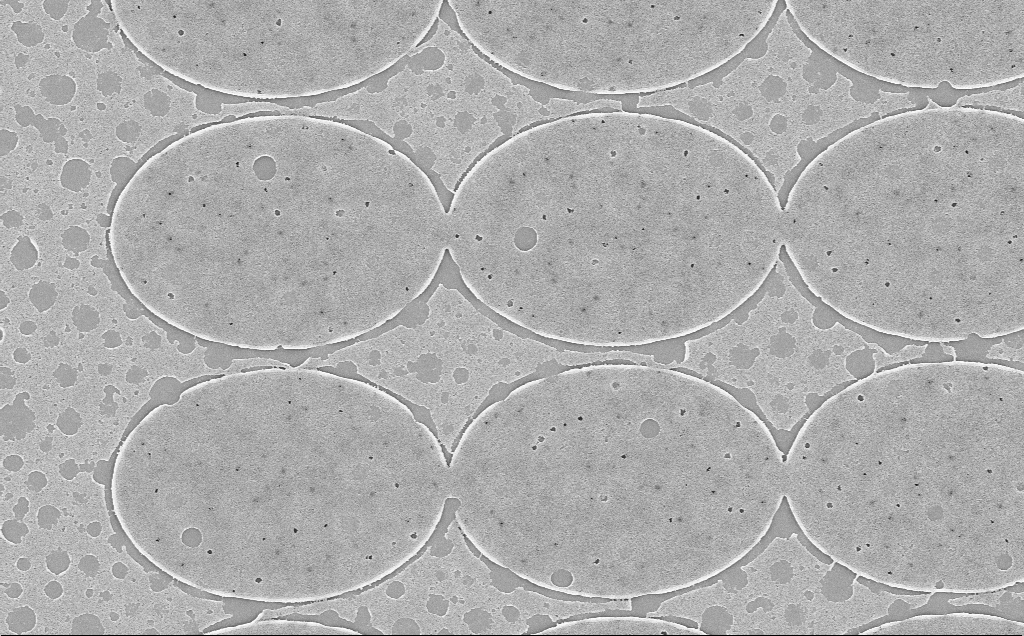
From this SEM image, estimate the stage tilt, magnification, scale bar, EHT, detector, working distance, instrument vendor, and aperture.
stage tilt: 0°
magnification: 12.37 K X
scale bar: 2000 nm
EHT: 5 kV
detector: InLens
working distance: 6 mm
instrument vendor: Zeiss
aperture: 30 µm